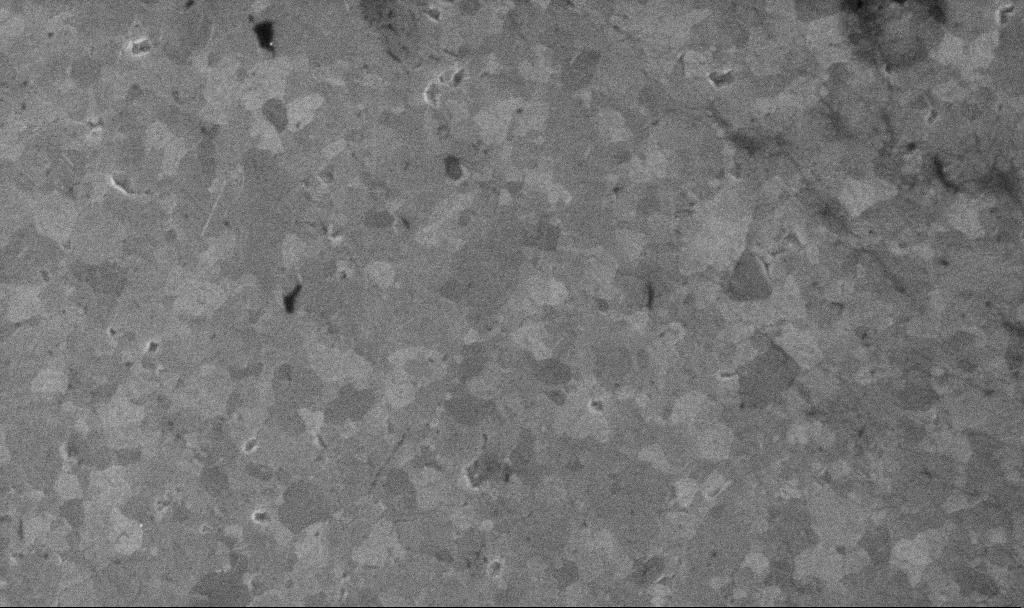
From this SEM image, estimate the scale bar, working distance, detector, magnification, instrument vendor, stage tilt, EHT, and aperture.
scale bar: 1000 nm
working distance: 3.1 mm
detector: InLens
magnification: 50.56 K X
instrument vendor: Zeiss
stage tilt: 0°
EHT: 10 kV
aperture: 30 µm